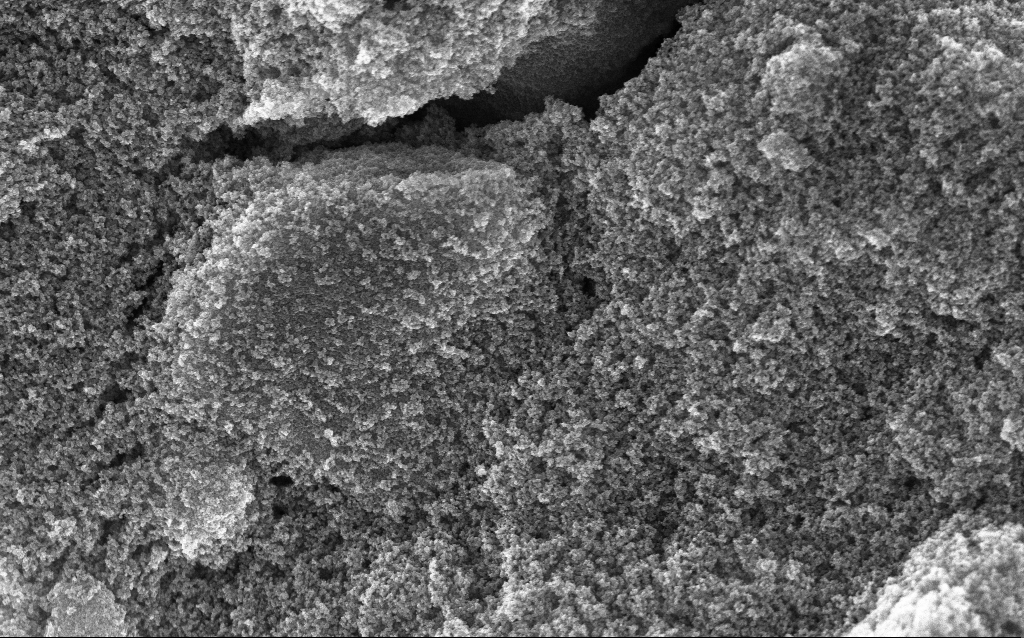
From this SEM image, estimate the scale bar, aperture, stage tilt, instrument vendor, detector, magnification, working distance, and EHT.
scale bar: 1000 nm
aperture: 30 µm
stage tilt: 0°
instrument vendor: Zeiss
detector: InLens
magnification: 37.88 K X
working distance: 2.6 mm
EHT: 10 kV